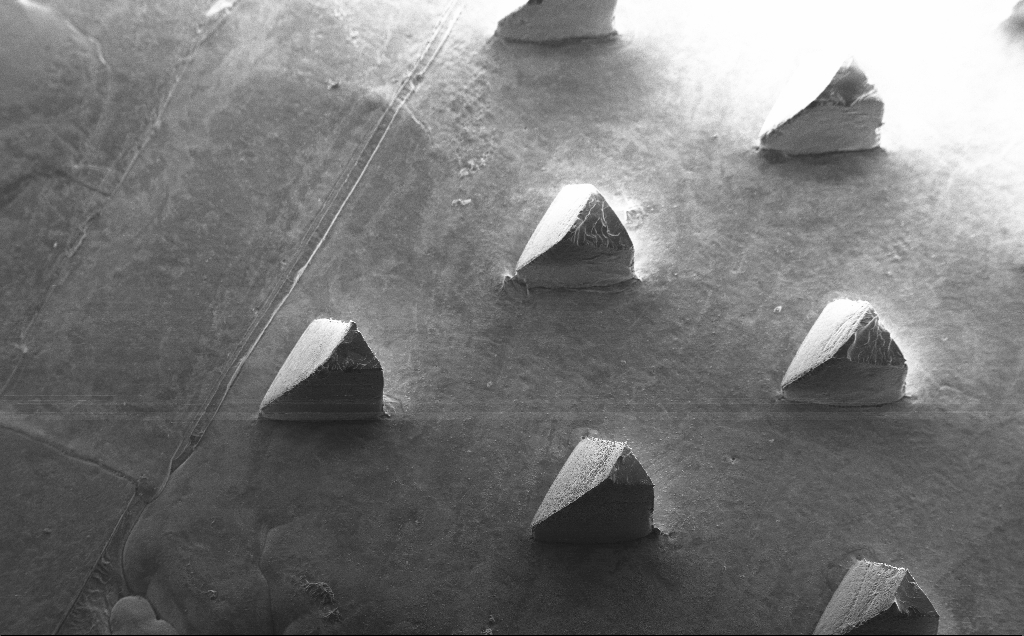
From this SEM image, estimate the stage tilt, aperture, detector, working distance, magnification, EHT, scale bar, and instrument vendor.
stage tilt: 30°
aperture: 30 µm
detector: SE2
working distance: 9 mm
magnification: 0.08 K X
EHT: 10 kV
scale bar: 200000 nm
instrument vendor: Zeiss